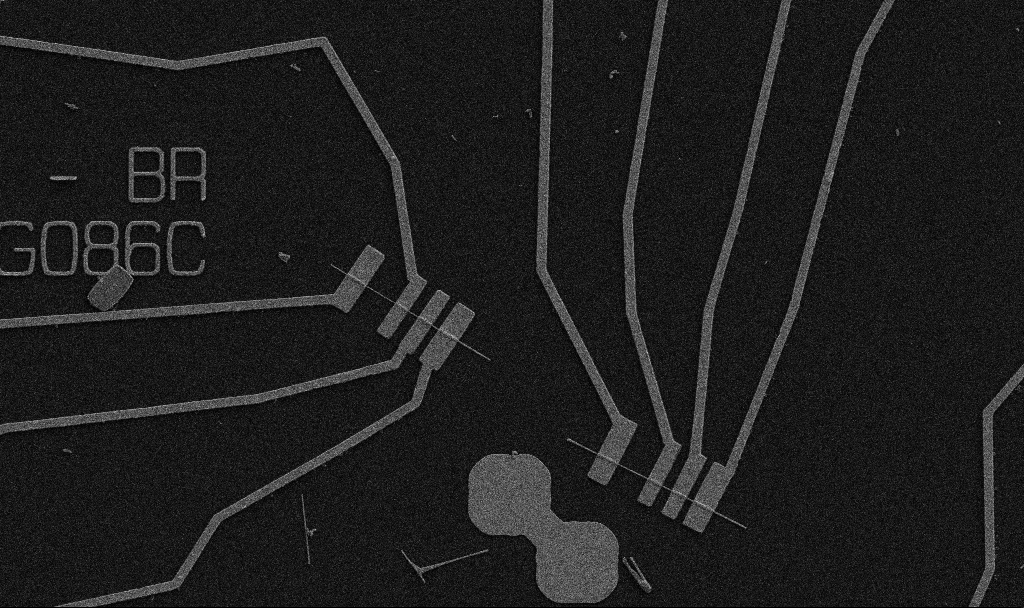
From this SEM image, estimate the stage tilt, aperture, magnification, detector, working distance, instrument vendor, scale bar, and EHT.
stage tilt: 0°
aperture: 30 µm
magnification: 5 K X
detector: SE2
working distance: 10.7 mm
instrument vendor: Zeiss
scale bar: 10000 nm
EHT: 5 kV